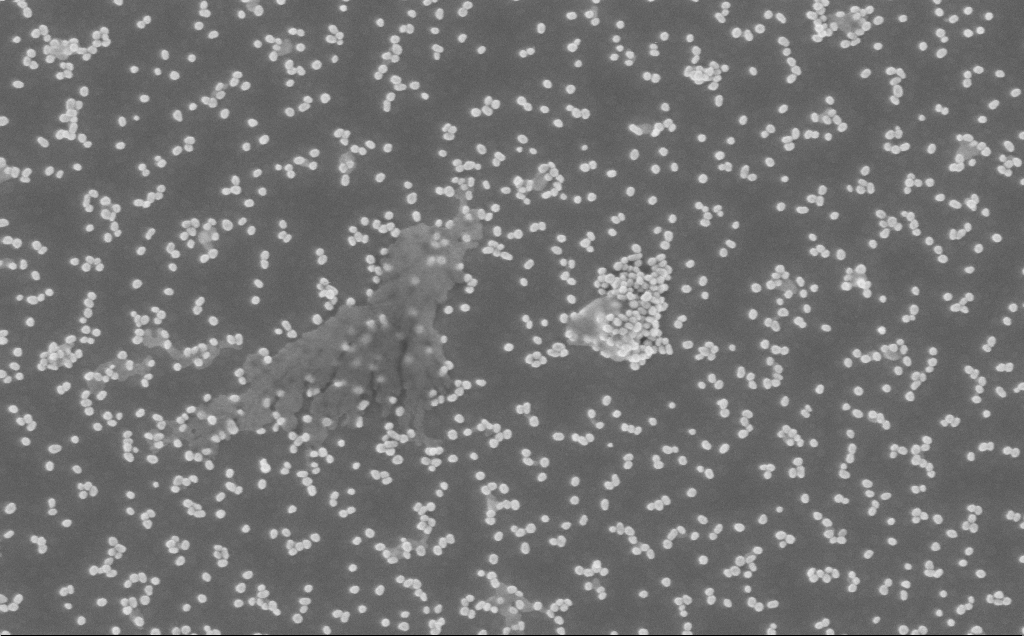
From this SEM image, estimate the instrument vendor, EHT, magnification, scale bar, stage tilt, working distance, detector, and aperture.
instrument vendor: Zeiss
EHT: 3 kV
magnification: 80 K X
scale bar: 200 nm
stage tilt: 0°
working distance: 5 mm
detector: InLens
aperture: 30 µm